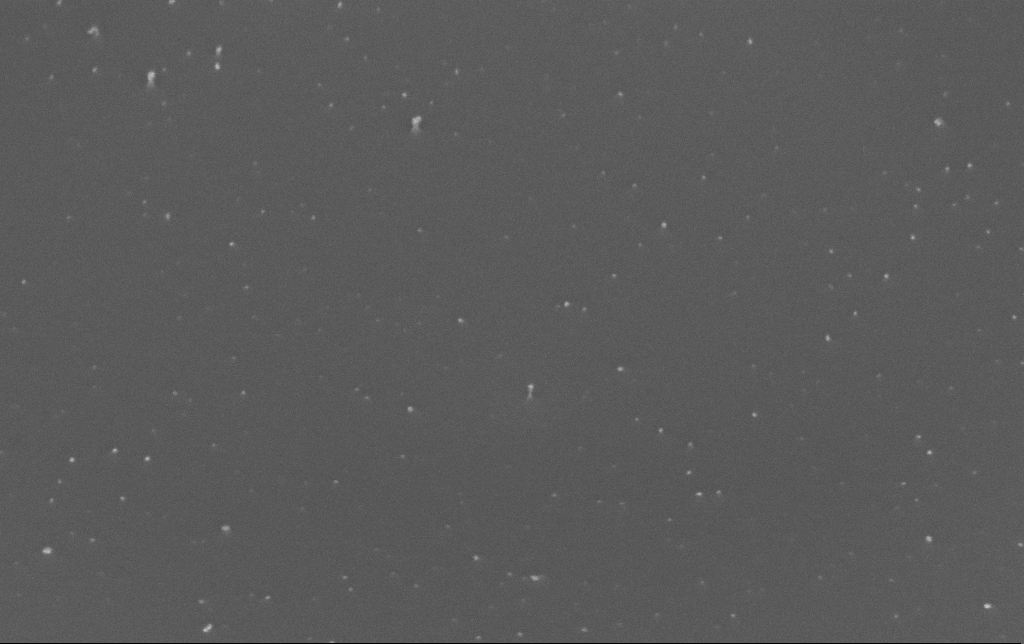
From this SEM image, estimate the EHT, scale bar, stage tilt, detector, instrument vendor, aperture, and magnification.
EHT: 10 kV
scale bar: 100 nm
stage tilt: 45°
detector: InLens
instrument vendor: Zeiss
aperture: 30 µm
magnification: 200 K X